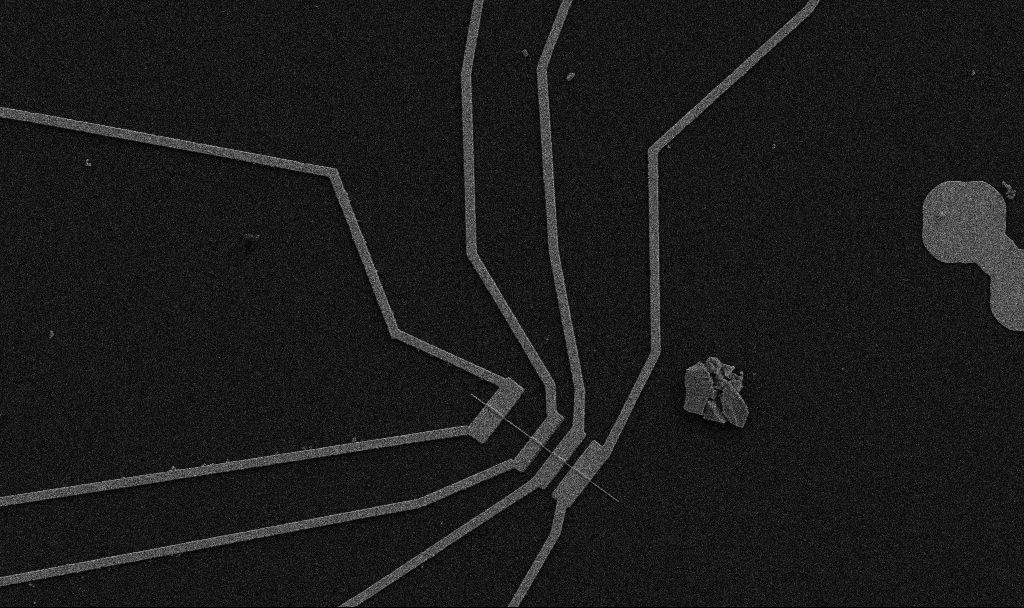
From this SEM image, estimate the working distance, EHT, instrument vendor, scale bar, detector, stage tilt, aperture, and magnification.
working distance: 10.7 mm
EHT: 5 kV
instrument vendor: Zeiss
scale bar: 10000 nm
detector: SE2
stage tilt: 0°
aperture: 30 µm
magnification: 5 K X